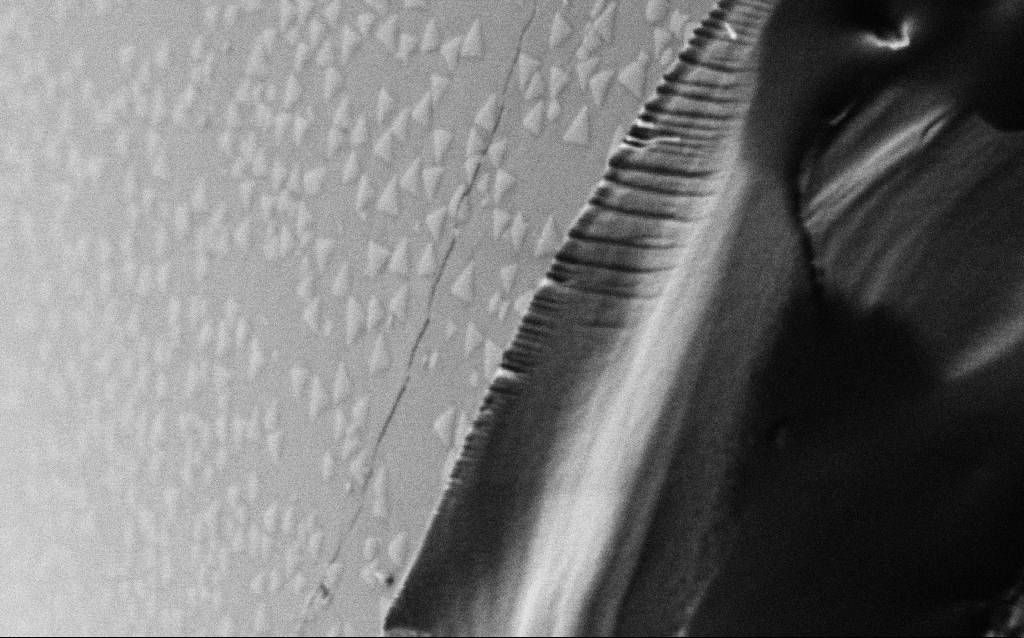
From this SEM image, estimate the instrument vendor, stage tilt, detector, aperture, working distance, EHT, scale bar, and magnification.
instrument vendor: Zeiss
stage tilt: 45°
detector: SE2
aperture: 30 µm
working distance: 6.6 mm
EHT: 1 kV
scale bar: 200 nm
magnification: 100 K X